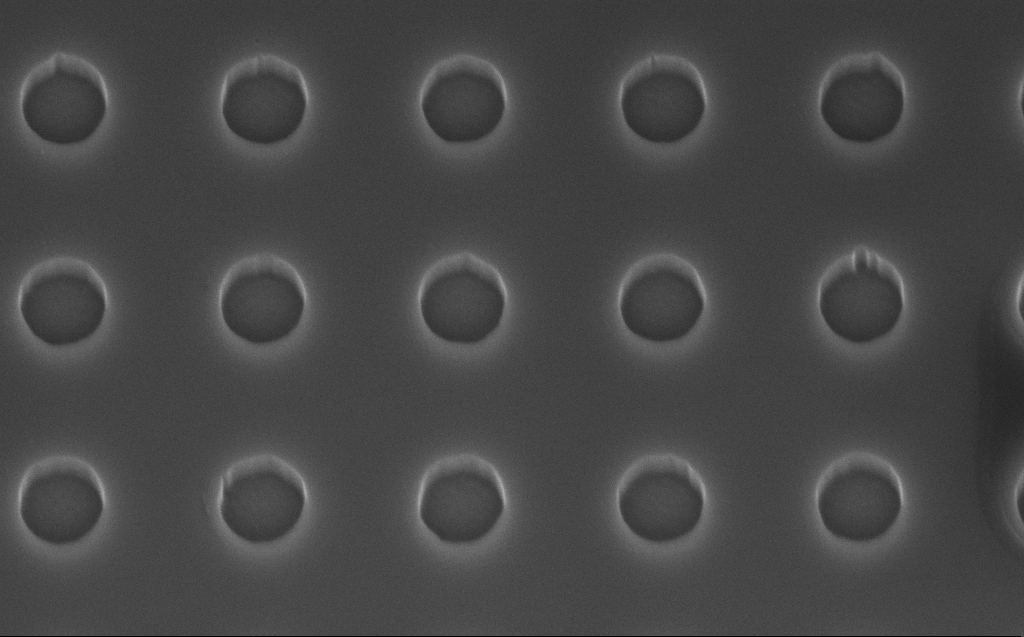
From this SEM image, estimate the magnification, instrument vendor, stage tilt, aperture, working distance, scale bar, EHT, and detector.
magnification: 69.88 K X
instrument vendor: Zeiss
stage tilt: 45°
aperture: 30 µm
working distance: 7 mm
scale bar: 1000 nm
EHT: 5 kV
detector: InLens